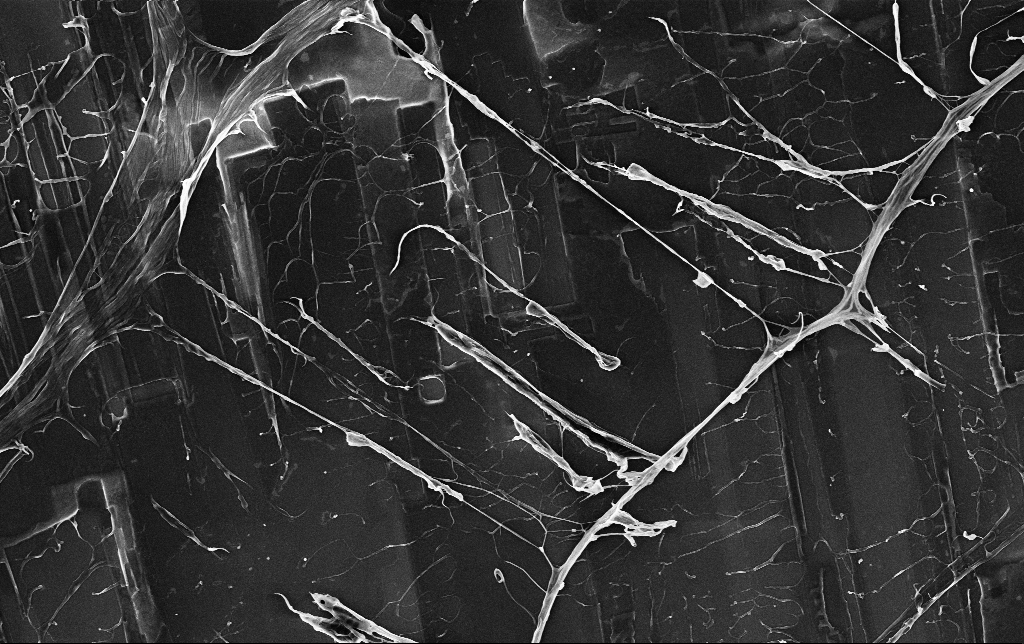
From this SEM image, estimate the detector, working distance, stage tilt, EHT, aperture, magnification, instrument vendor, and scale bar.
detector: InLens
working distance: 3.5 mm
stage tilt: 0°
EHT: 3 kV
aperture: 30 µm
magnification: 6.83 K X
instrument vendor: Zeiss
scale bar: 10000 nm